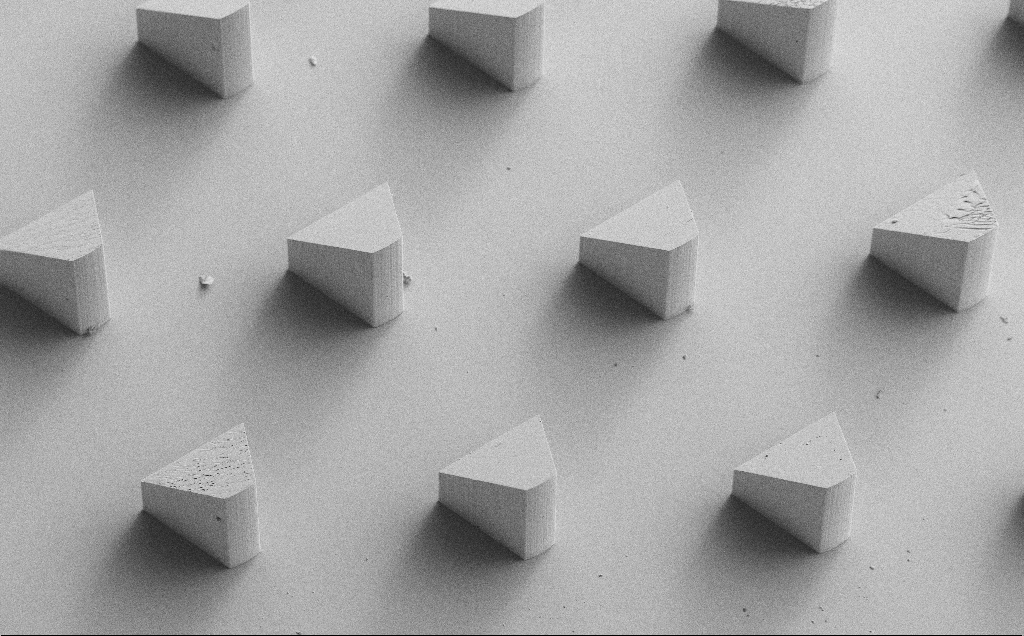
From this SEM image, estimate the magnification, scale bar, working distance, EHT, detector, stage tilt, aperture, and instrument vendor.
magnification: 0.111 K X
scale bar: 200000 nm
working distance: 9 mm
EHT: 10 kV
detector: SE2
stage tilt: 20°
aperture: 30 µm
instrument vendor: Zeiss